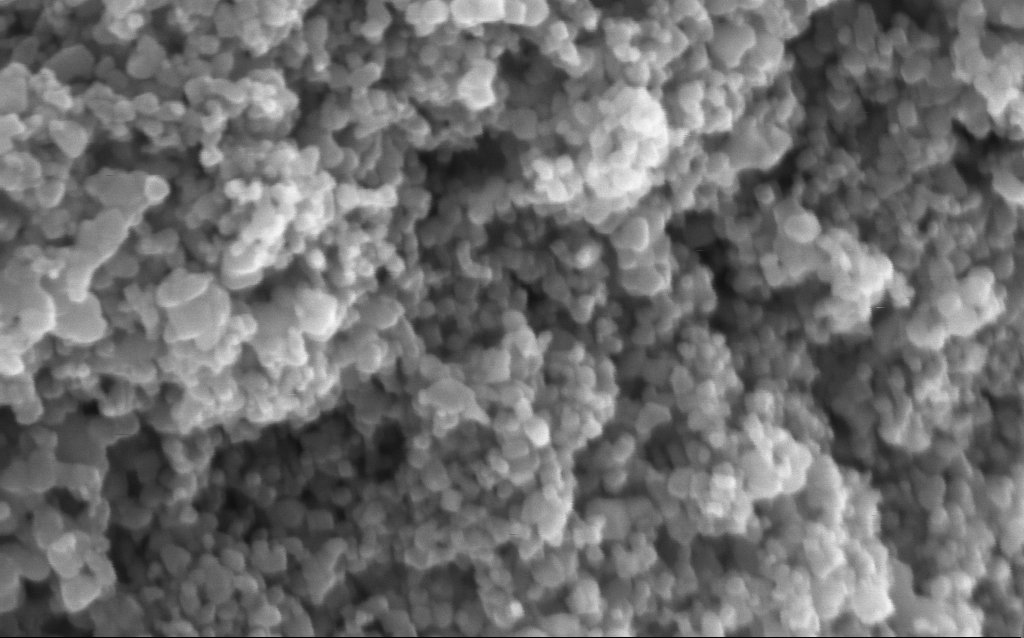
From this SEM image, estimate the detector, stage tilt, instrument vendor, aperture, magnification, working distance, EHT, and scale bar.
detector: InLens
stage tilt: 0°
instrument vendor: Zeiss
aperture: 30 µm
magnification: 282.06 K X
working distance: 4 mm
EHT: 5 kV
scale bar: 200 nm